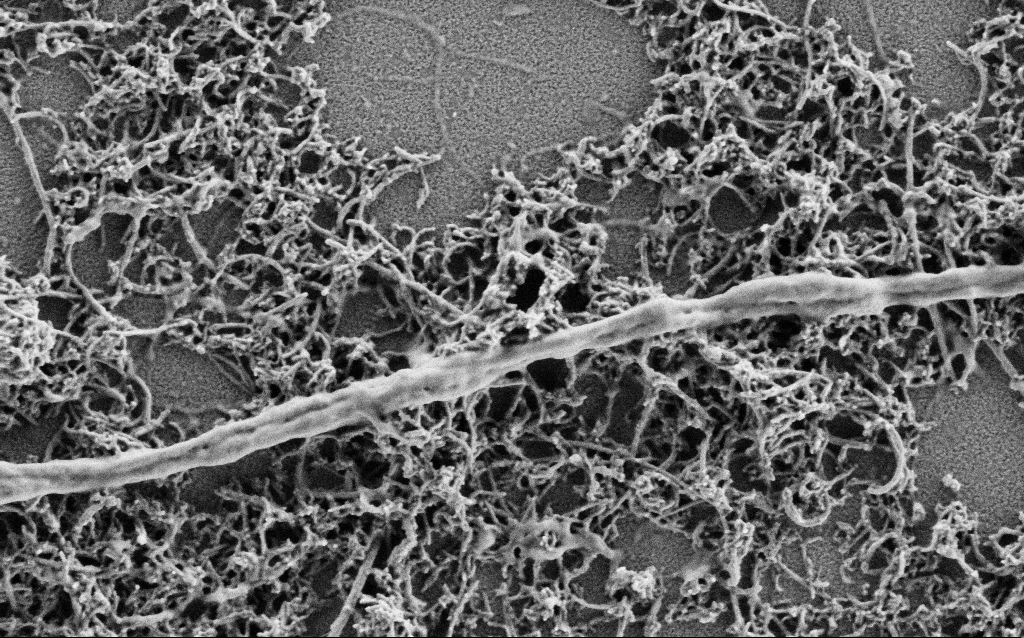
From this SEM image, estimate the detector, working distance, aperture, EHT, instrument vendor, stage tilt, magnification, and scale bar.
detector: SE2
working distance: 4 mm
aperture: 30 µm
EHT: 1 kV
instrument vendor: Zeiss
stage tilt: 0°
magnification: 50 K X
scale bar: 1000 nm